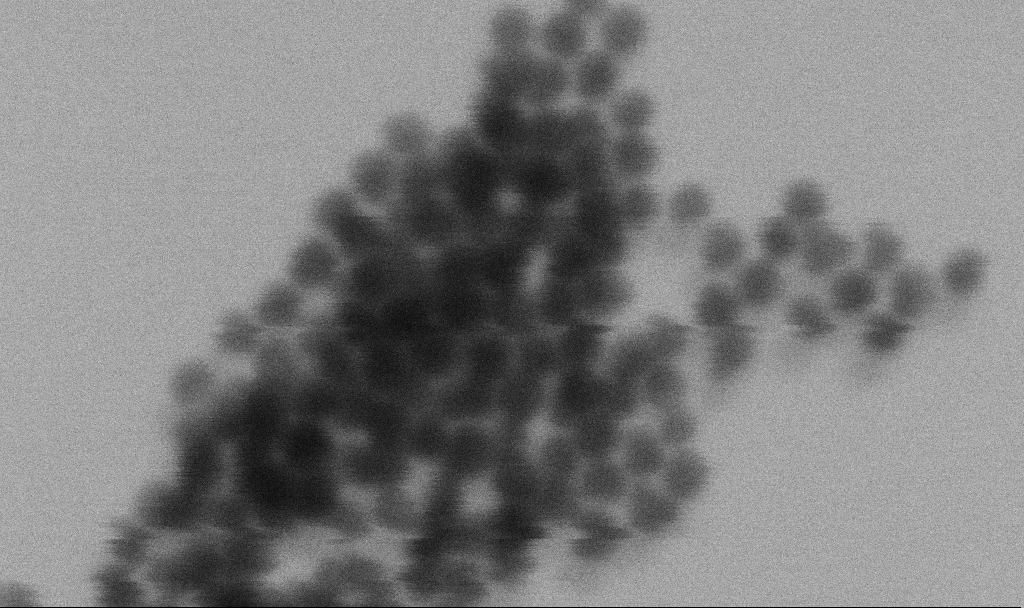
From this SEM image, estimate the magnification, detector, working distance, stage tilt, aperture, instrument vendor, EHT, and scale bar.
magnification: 969.43 K X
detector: SE2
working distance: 6 mm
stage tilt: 0°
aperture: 30 µm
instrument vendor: Zeiss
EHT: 6 kV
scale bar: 20 nm